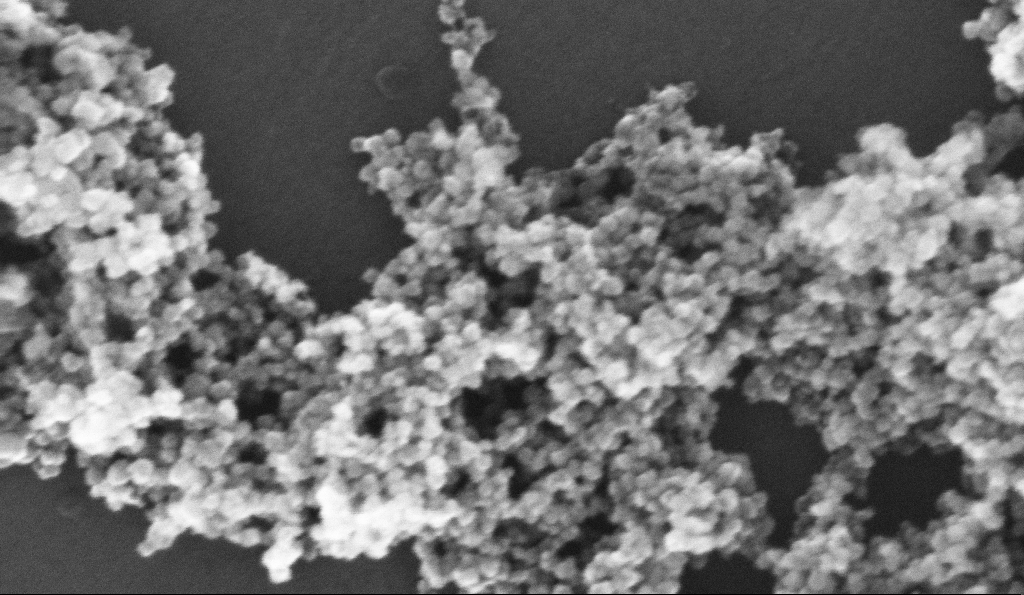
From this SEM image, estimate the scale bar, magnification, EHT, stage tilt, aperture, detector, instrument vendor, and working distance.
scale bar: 100 nm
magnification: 363.47 K X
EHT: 10 kV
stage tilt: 0°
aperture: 30 µm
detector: InLens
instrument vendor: Zeiss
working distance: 5.2 mm